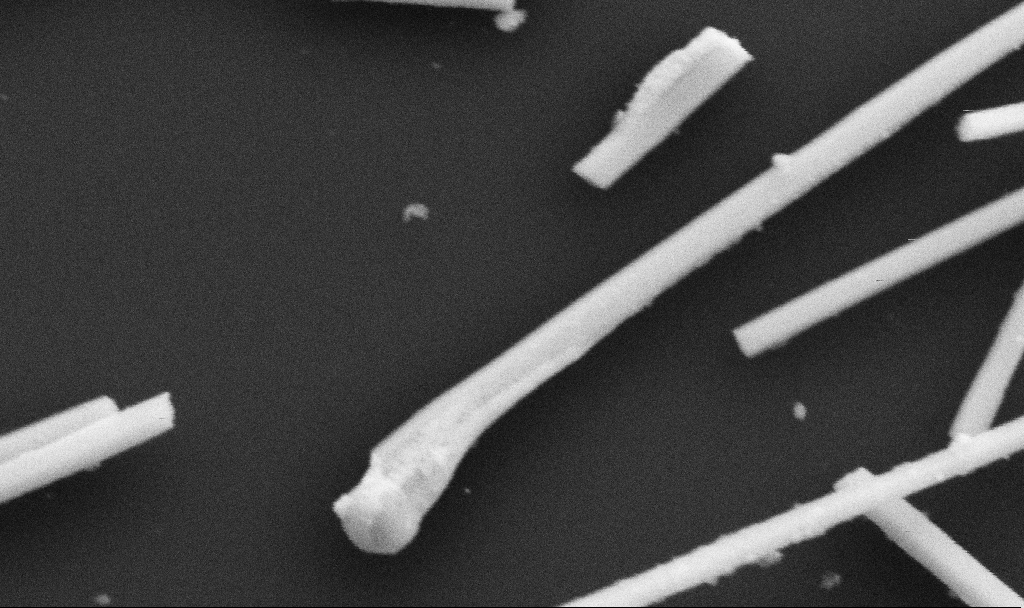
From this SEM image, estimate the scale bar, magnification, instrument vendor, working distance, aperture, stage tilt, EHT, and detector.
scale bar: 200 nm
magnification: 132.69 K X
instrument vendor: Zeiss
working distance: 10.7 mm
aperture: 30 µm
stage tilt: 0°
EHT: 5 kV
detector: SE2